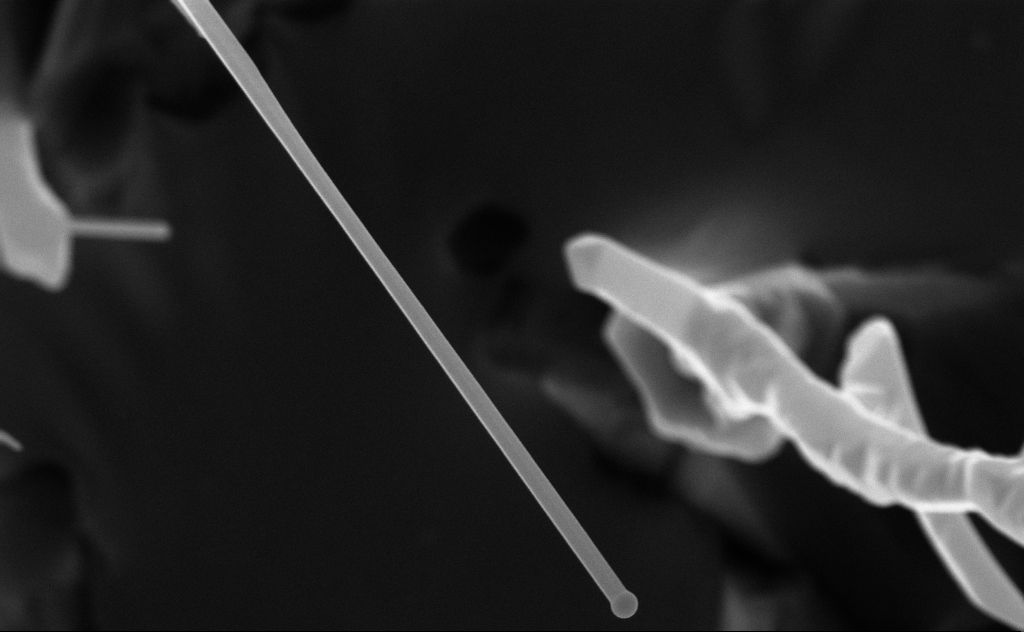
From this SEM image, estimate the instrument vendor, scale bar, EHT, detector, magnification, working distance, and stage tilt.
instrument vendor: Zeiss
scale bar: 200 nm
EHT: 10 kV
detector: InLens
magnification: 74.46 K X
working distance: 6 mm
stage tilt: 0°